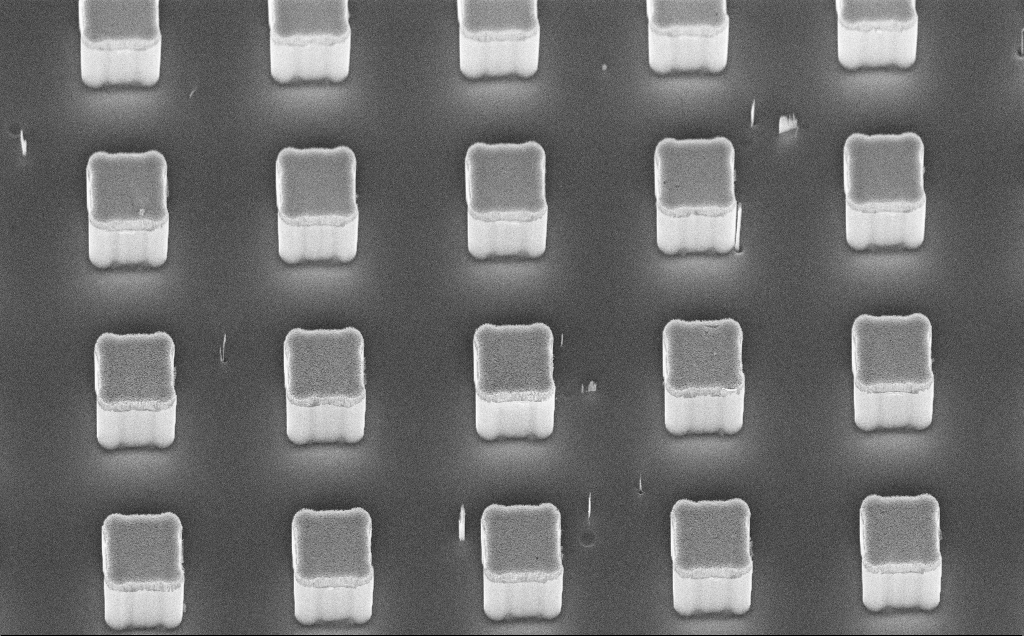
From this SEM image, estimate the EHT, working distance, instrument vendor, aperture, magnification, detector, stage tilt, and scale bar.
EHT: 10 kV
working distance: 11 mm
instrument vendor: Zeiss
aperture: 30 µm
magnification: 3.48 K X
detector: InLens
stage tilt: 45°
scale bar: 10000 nm